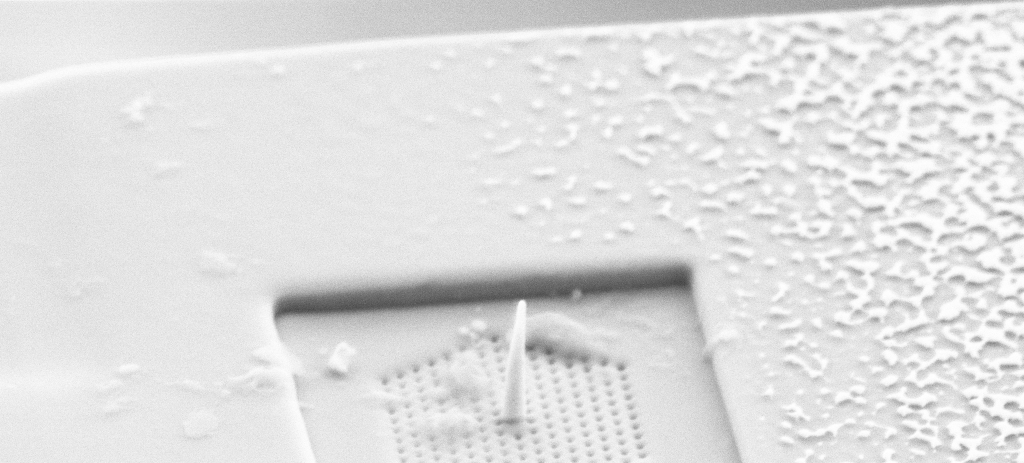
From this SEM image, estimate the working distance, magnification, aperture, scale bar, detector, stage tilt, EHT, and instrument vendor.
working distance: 6 mm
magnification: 25.28 K X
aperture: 30 µm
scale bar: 1000 nm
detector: SE2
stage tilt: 53.9°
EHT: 5 kV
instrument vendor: Zeiss